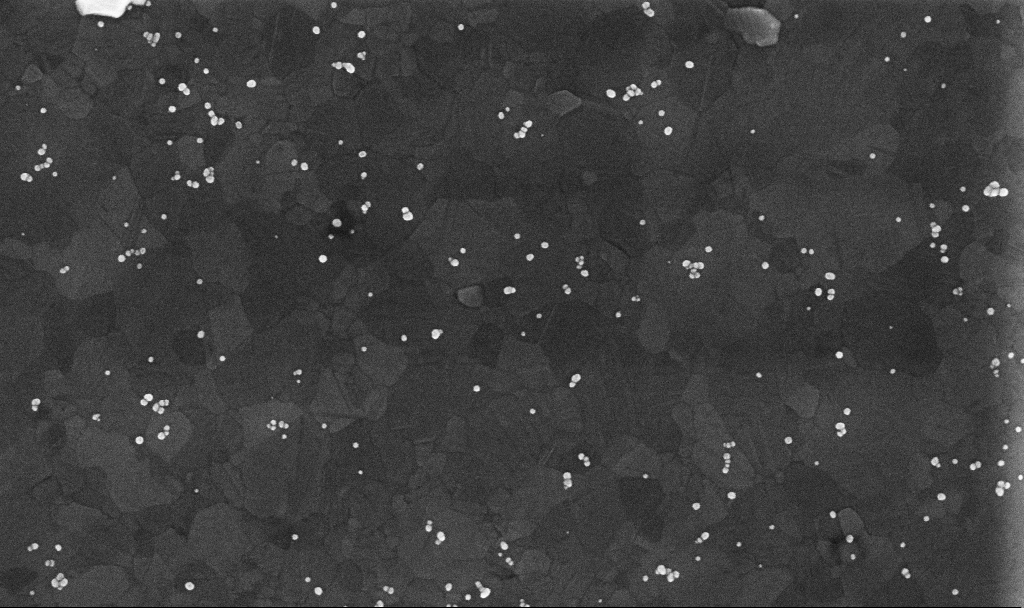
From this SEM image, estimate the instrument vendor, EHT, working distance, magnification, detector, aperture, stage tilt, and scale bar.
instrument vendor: Zeiss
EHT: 10 kV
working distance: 3.4 mm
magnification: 100.84 K X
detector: InLens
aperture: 30 µm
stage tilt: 0°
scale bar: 200 nm